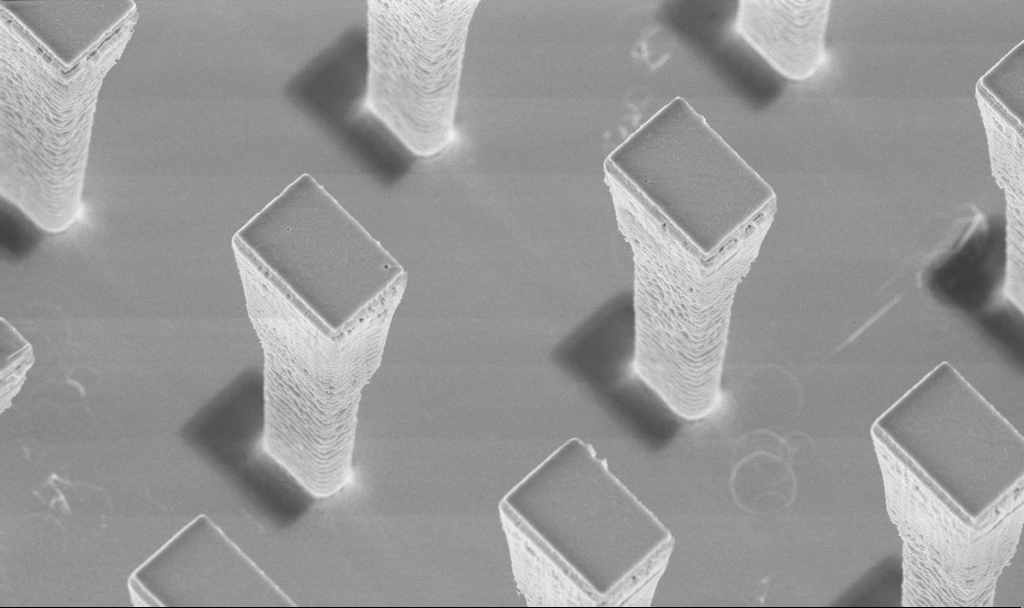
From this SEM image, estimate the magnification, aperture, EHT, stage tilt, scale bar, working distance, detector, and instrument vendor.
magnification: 11.94 K X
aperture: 30 µm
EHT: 5 kV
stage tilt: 20°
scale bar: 2000 nm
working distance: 4.2 mm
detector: InLens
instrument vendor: Zeiss